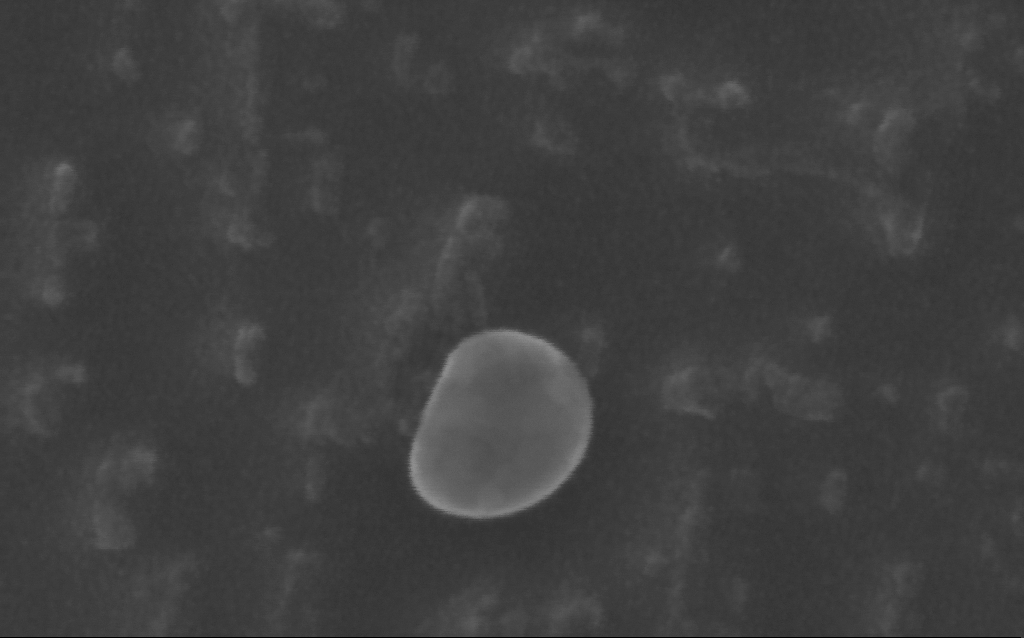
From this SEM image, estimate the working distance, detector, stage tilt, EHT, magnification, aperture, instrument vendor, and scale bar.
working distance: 5 mm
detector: InLens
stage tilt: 0°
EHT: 10 kV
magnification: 655.23 K X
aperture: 30 µm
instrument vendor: Zeiss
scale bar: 100 nm